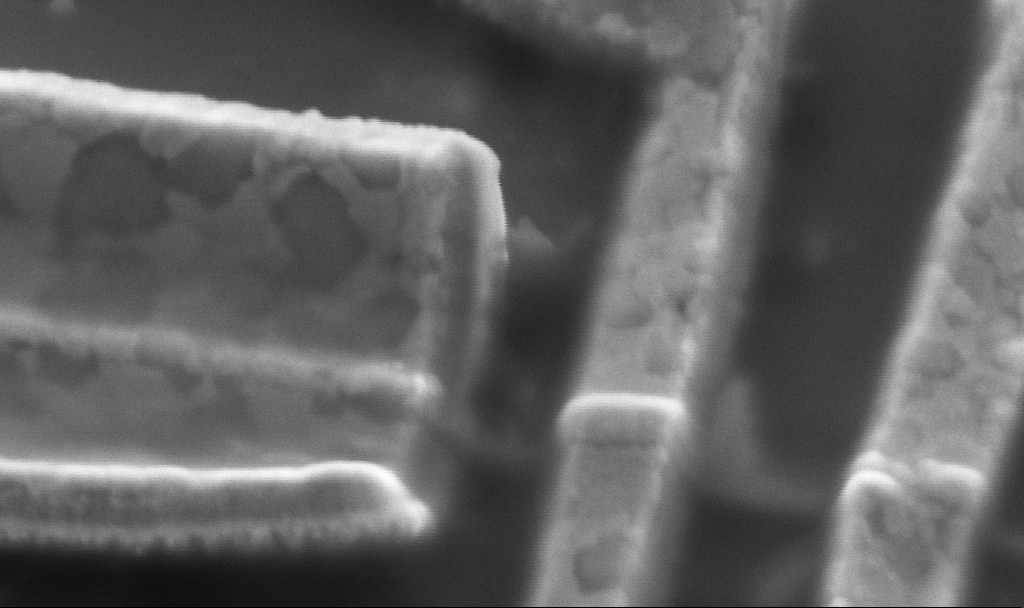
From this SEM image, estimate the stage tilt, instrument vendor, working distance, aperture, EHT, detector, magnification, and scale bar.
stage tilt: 45°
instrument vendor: Zeiss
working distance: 16.7 mm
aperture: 30 µm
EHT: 5 kV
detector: SE2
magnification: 100 K X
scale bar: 200 nm